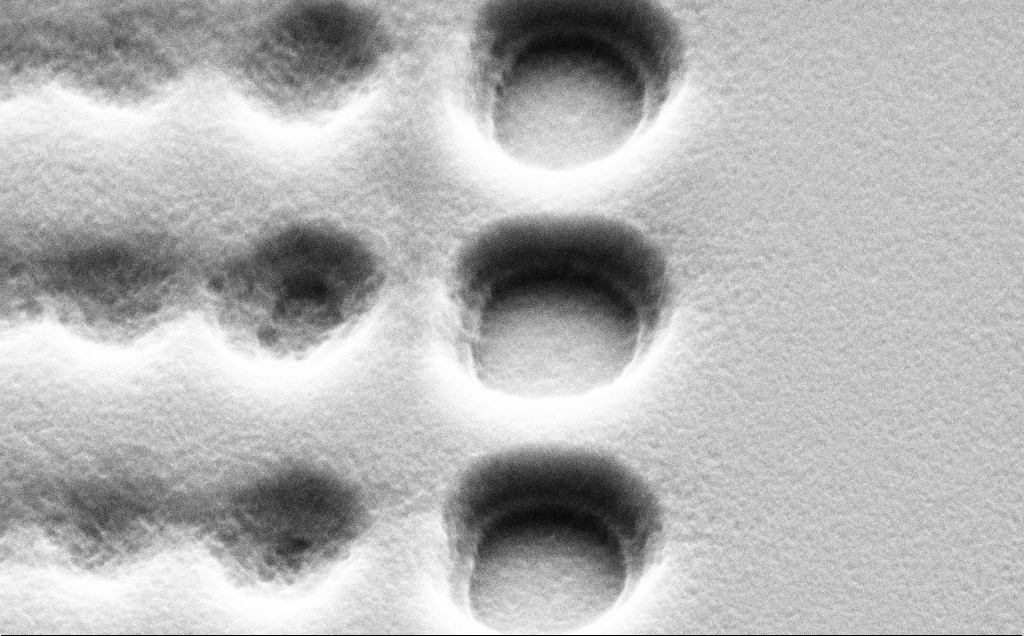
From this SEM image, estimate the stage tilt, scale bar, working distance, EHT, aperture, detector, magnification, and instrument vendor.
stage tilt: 45°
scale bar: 1000 nm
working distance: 10 mm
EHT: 5 kV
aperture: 30 µm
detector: SE2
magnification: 43.87 K X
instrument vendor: Zeiss